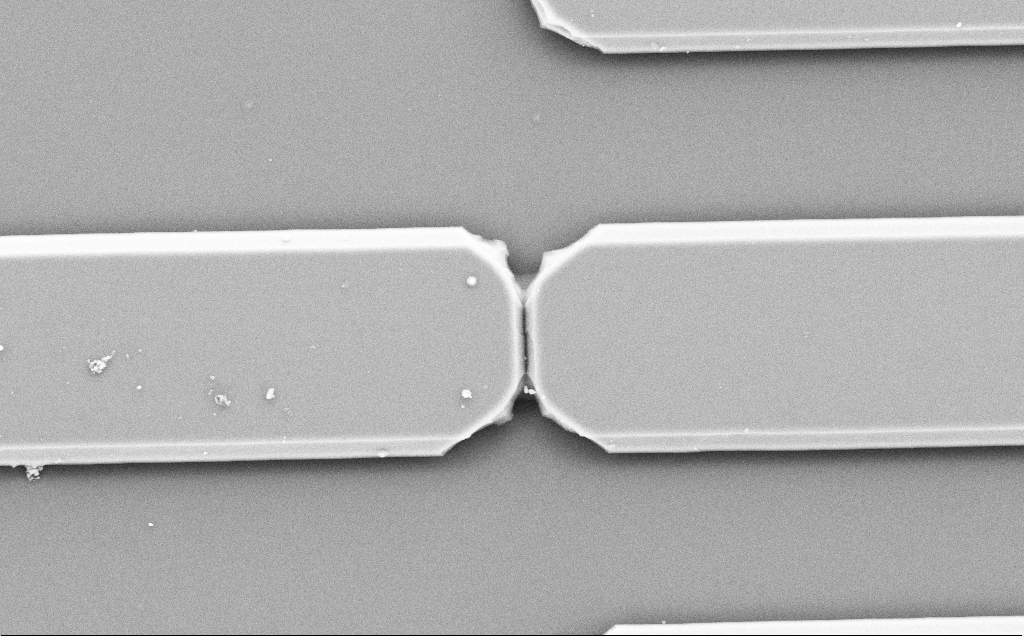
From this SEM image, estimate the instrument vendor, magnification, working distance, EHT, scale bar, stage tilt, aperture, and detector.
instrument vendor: Zeiss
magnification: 3.73 K X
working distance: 10 mm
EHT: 10 kV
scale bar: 10000 nm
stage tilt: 0°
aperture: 30 µm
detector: SE2